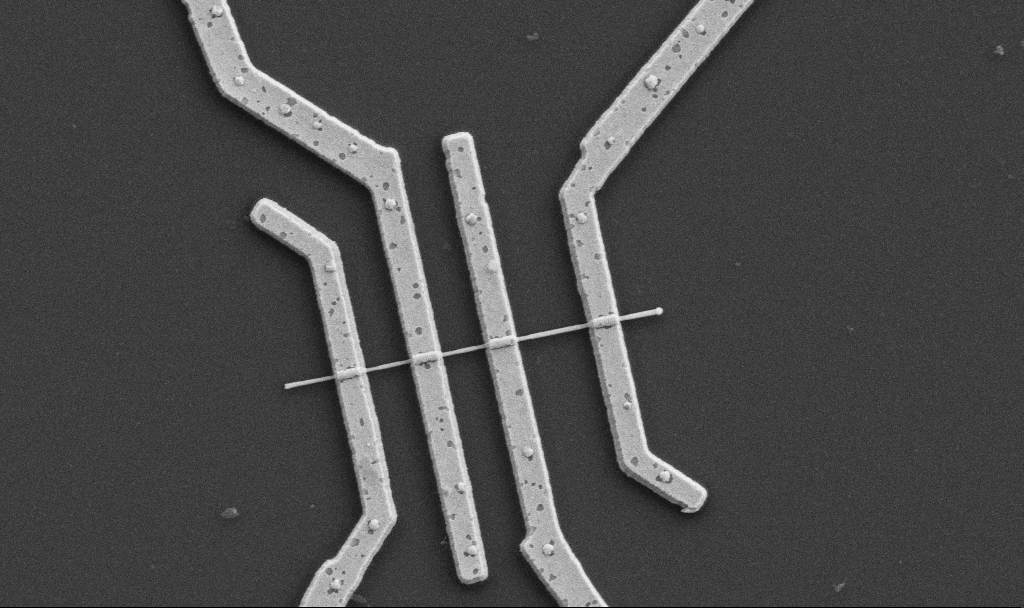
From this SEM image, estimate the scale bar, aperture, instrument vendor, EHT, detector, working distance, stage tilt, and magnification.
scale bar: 1000 nm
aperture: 30 µm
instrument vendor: Zeiss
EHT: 5 kV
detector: SE2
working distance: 10.6 mm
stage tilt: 0°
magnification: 20 K X